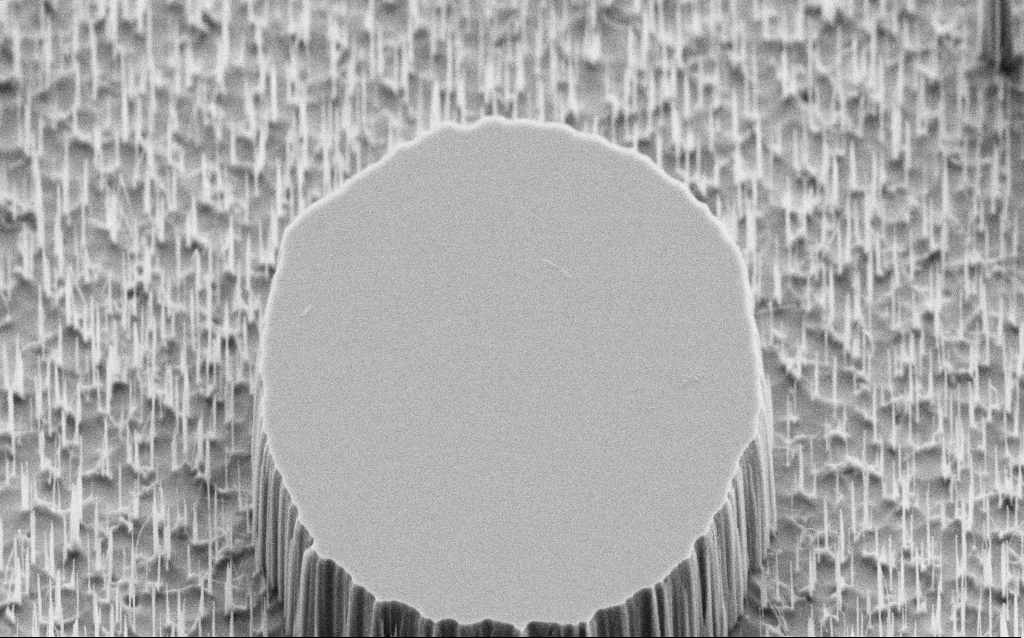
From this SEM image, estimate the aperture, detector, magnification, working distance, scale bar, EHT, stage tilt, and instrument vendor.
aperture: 30 µm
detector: SE2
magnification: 10 K X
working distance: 7 mm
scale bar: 2000 nm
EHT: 5 kV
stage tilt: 45°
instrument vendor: Zeiss